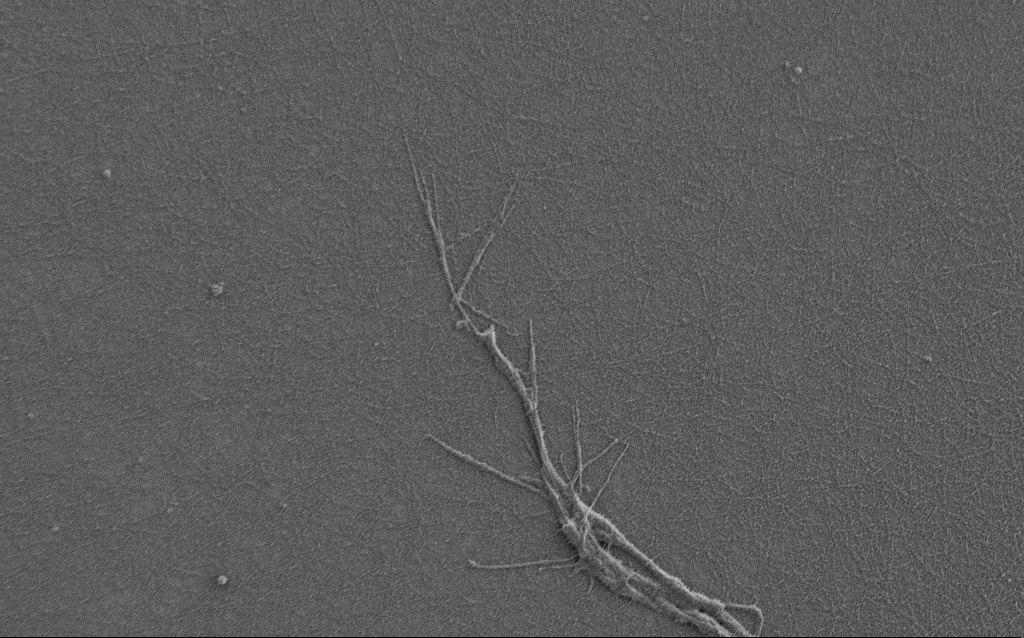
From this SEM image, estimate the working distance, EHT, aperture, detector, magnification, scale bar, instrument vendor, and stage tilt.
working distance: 6 mm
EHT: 0.9 kV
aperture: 30 µm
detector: SE2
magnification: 7.5 K X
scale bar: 2000 nm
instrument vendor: Zeiss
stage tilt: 0°